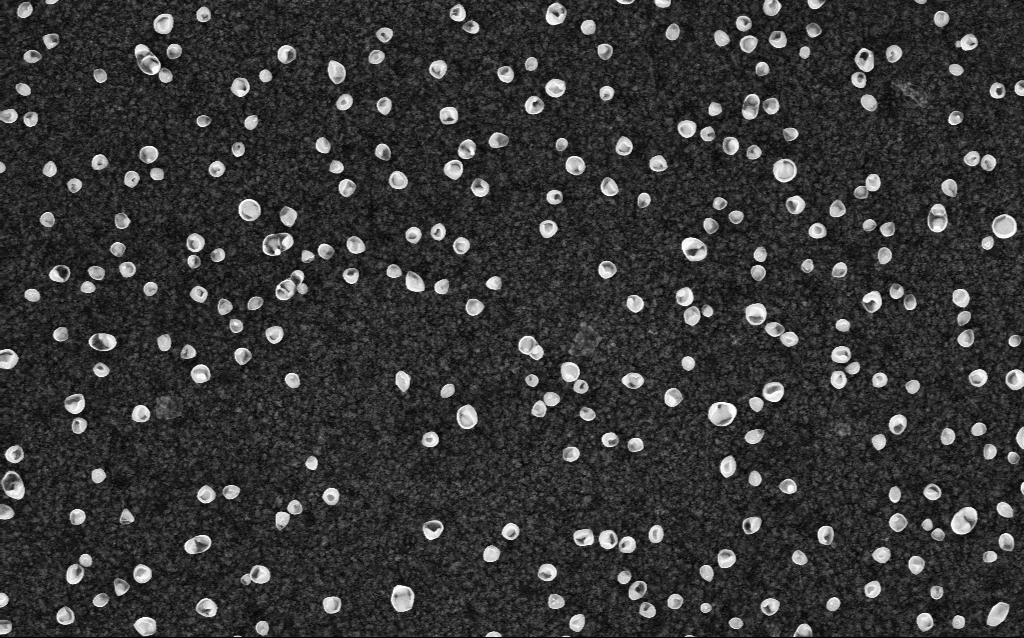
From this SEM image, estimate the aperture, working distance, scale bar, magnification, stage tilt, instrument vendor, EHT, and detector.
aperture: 30 µm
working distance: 2.8 mm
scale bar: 1000 nm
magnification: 50 K X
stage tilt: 0°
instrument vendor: Zeiss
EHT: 5 kV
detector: InLens